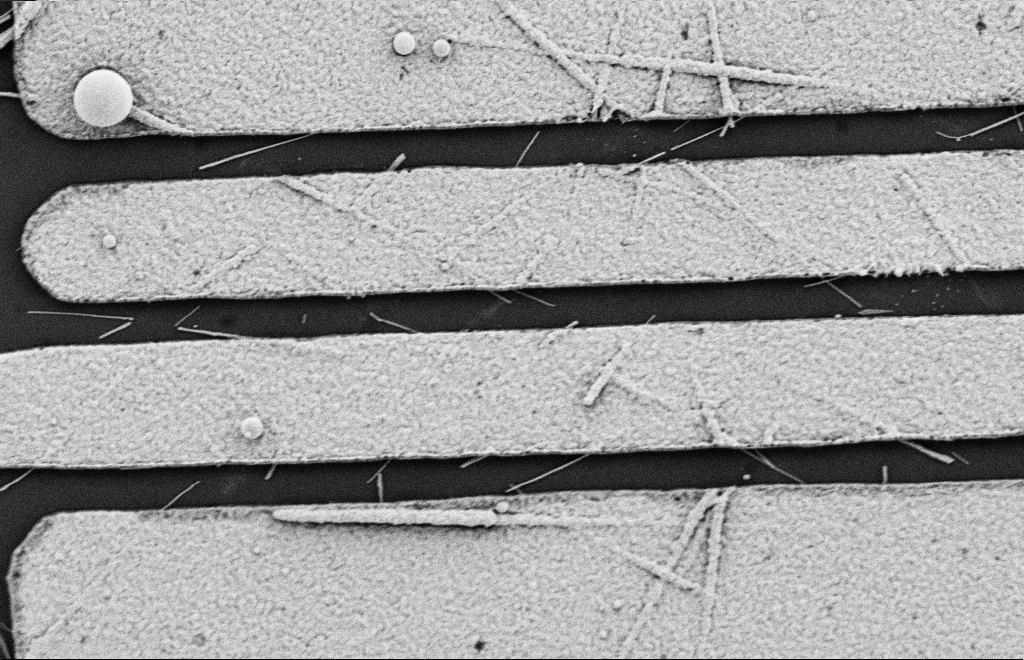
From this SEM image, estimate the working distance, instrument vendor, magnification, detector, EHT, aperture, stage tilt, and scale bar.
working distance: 9 mm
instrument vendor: Zeiss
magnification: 16.45 K X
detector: SE2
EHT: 2 kV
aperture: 20 µm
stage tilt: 0°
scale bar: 2000 nm